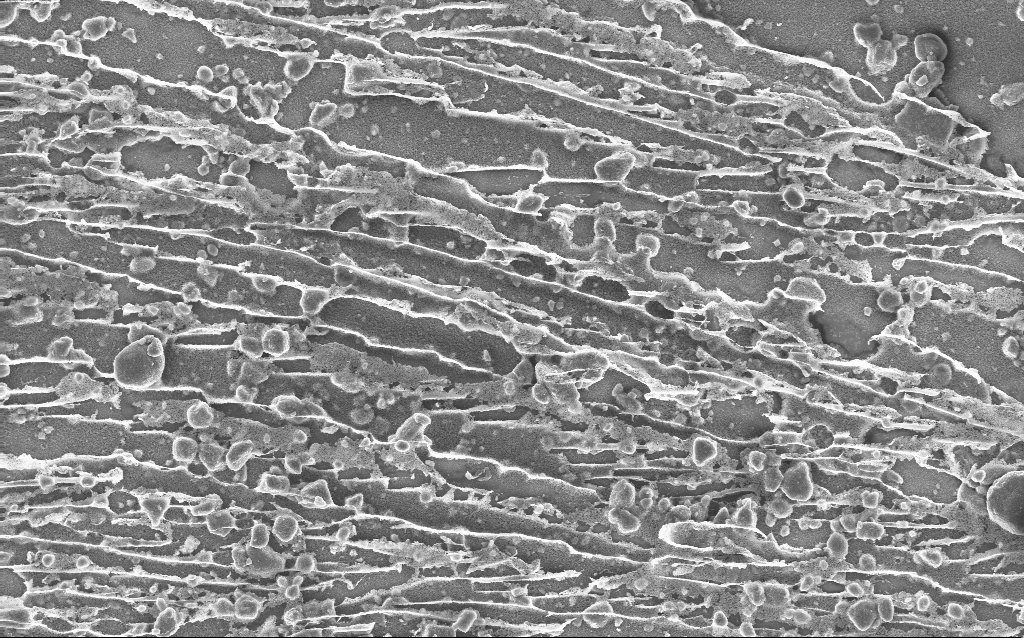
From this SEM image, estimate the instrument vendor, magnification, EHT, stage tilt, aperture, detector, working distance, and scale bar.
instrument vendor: Zeiss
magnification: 1.69 K X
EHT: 10 kV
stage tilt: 0°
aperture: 30 µm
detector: InLens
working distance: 2.5 mm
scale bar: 10000 nm